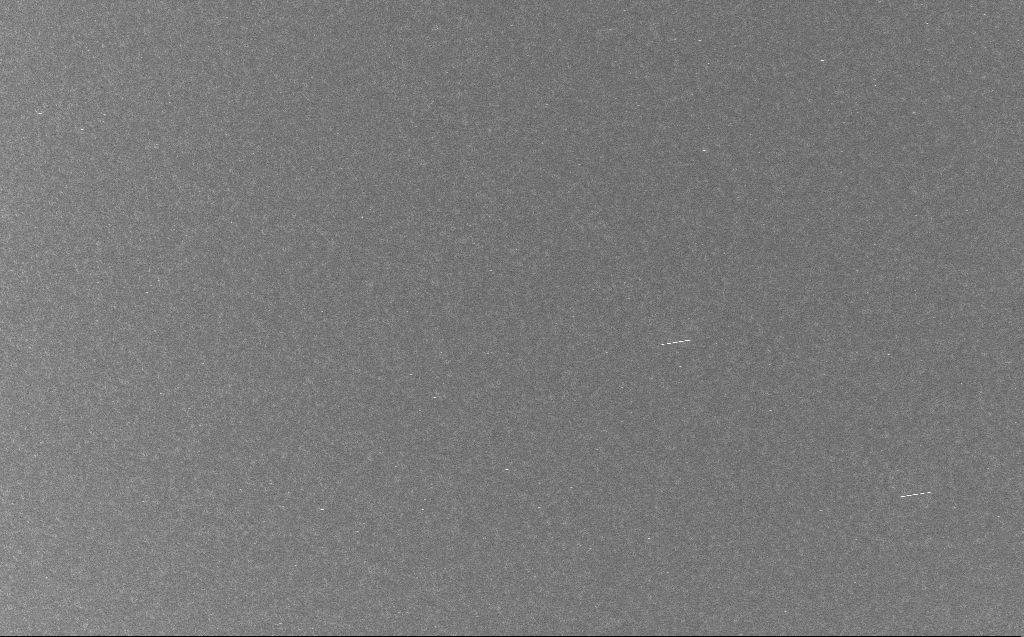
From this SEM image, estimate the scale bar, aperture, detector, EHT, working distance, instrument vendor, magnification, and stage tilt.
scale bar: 20000 nm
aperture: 30 µm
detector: InLens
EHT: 10 kV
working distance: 3 mm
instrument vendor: Zeiss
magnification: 2 K X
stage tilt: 0°